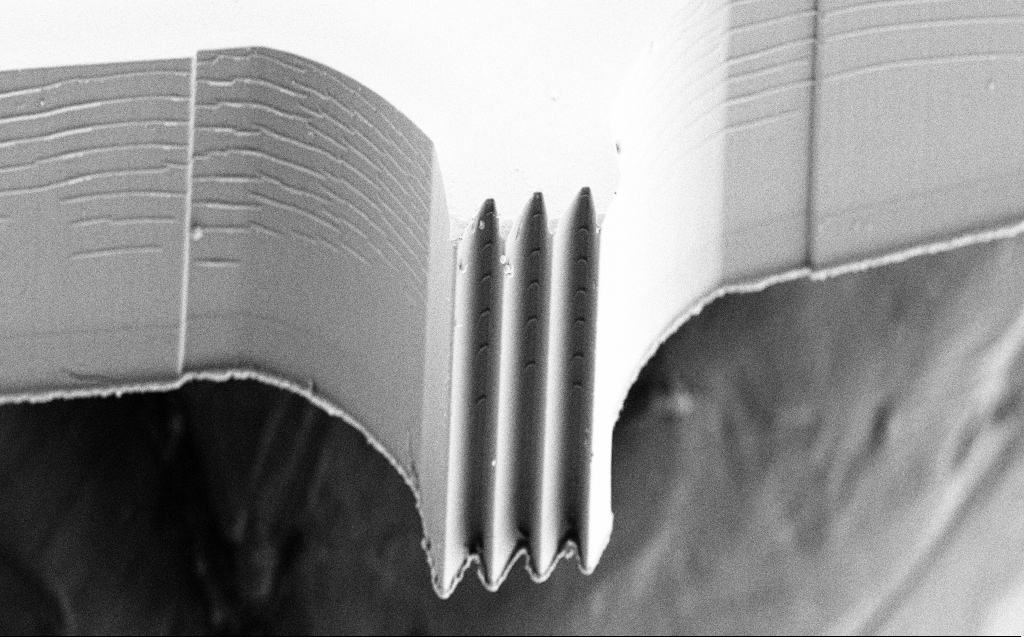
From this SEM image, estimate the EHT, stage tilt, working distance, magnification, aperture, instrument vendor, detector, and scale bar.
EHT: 10 kV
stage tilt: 45°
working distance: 5 mm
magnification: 1.6 K X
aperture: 30 µm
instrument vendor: Zeiss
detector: SE2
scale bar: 10000 nm